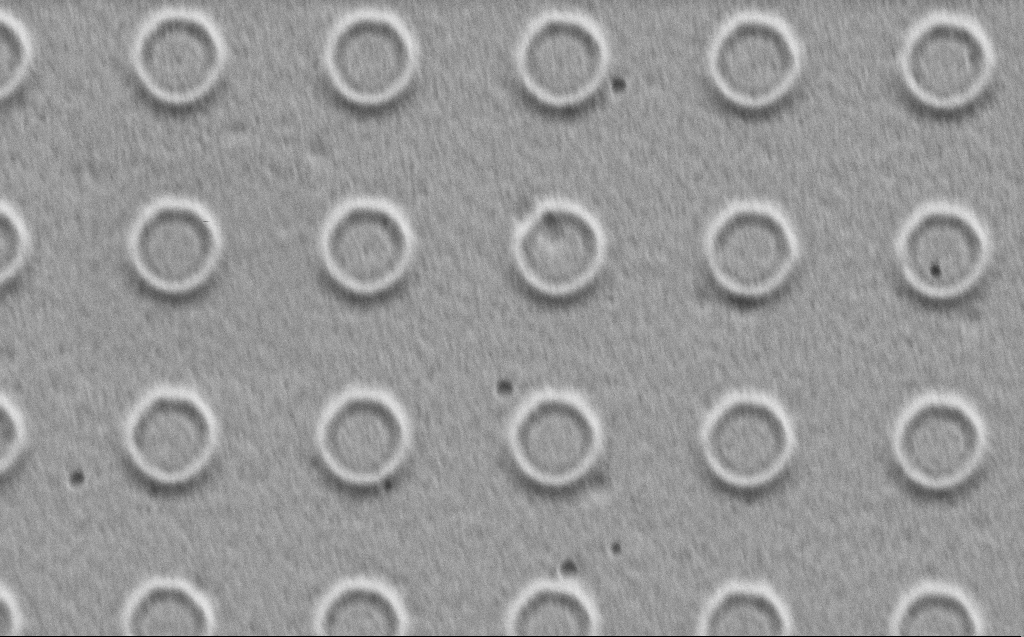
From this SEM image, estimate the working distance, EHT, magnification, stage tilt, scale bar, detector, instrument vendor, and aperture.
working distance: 4 mm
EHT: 1 kV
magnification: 59.31 K X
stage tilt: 30°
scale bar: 1000 nm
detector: SE2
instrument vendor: Zeiss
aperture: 30 µm